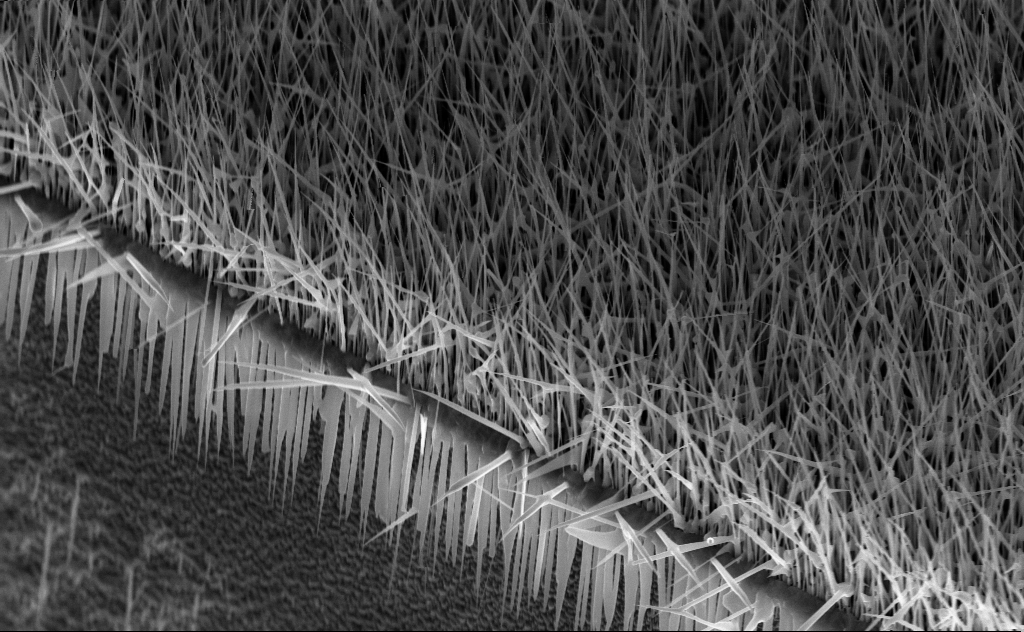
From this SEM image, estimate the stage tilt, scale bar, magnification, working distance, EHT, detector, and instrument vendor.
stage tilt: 44.2°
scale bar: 2000 nm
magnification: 20 K X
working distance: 7 mm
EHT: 10 kV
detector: InLens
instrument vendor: Zeiss